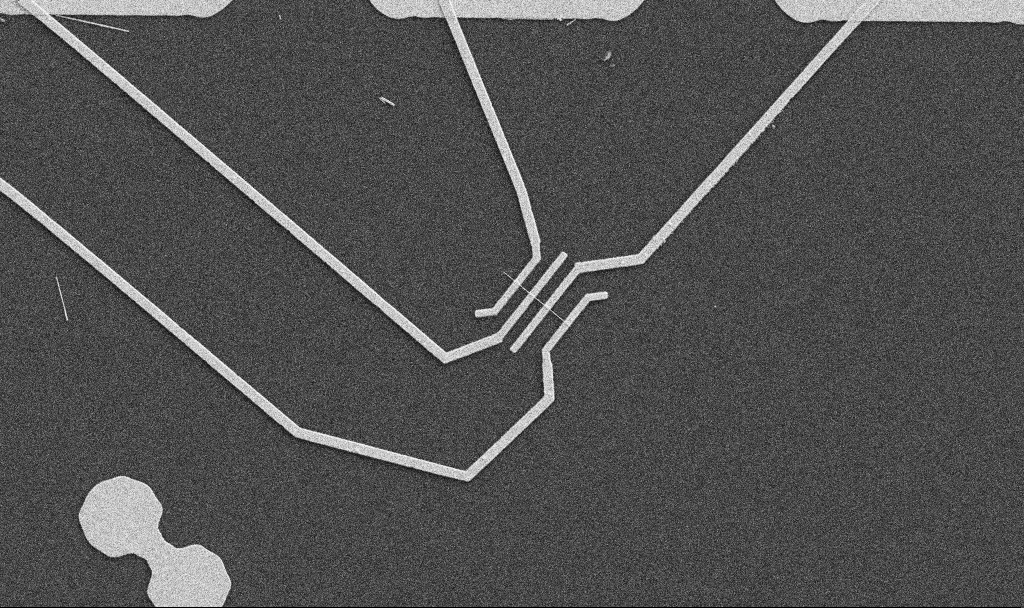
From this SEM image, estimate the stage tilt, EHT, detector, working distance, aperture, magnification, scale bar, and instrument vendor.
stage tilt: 0°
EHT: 5 kV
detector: SE2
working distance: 10.7 mm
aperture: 30 µm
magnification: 5 K X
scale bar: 10000 nm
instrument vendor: Zeiss